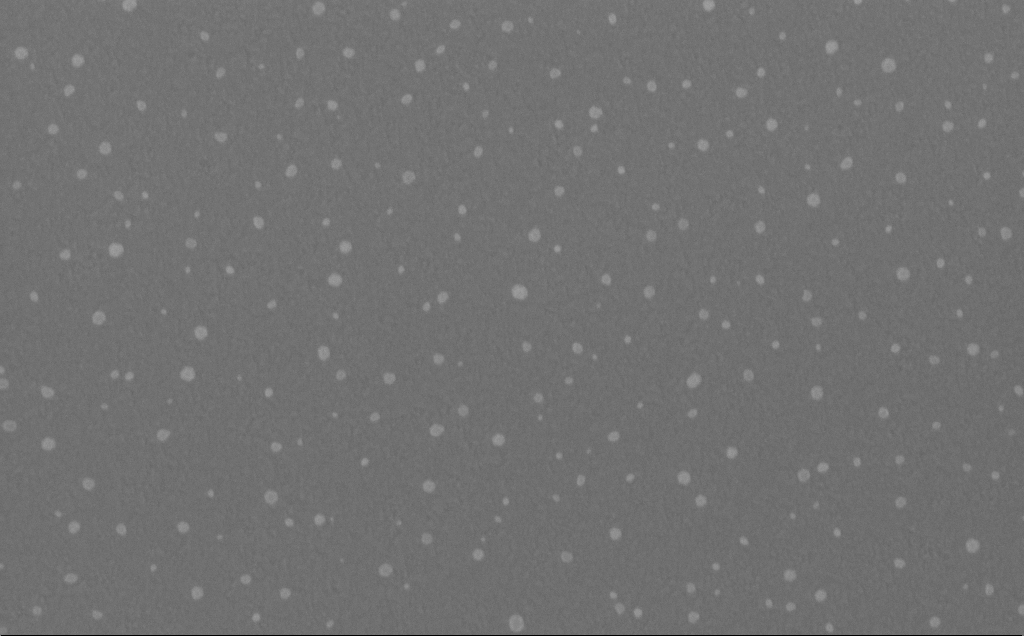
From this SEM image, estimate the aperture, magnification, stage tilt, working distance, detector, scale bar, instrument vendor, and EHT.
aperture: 30 µm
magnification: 80 K X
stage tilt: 0°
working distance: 5 mm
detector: InLens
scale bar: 200 nm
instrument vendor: Zeiss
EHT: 10 kV